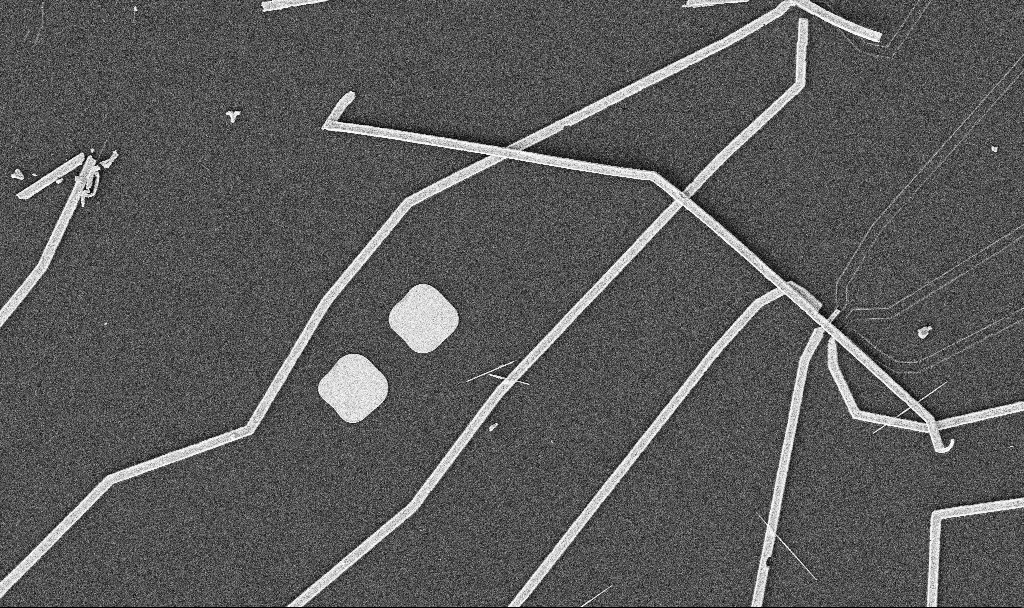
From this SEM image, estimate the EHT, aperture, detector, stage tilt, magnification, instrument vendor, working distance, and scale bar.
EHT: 5 kV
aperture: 30 µm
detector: SE2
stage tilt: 0°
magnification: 5 K X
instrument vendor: Zeiss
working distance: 10.7 mm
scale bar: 10000 nm